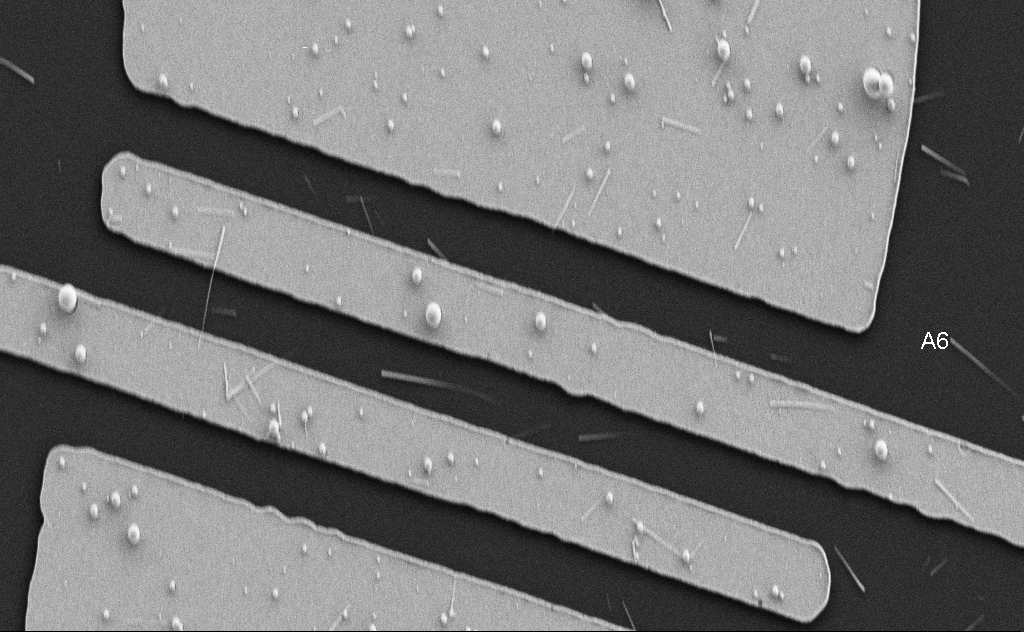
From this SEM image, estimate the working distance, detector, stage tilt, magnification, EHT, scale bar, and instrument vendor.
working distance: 5 mm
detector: SE2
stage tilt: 0°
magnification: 10.02 K X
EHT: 5 kV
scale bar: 2000 nm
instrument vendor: Zeiss